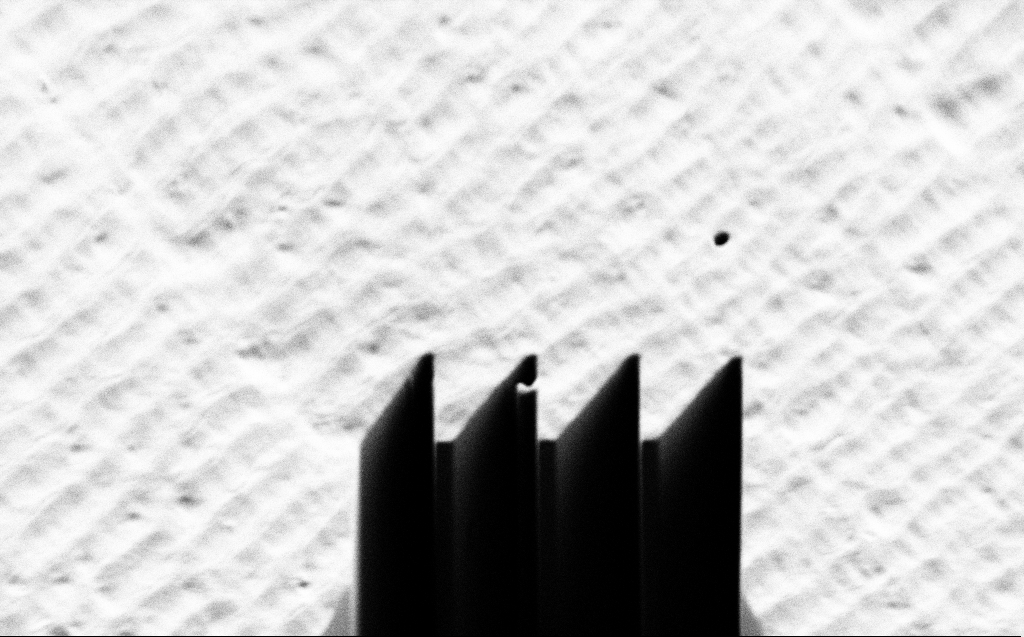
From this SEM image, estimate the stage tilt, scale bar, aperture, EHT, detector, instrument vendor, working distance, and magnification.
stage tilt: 45°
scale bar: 20000 nm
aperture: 30 µm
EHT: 1 kV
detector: SE2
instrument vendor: Zeiss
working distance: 6 mm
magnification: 3.33 K X